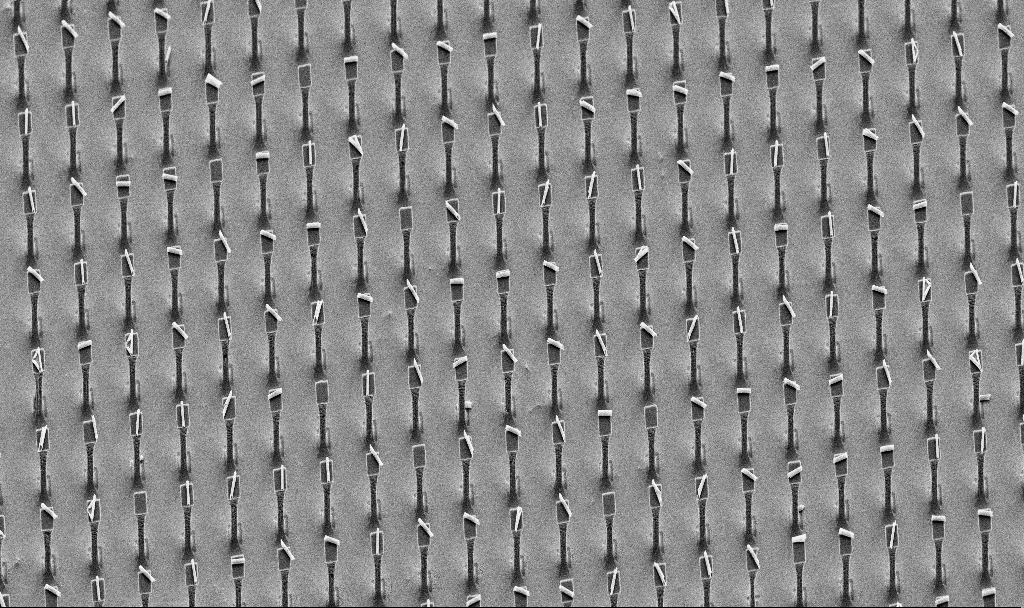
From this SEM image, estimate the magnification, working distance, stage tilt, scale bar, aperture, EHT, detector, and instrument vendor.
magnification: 1.42 K X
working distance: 9.5 mm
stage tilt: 30°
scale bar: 10000 nm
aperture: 30 µm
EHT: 5 kV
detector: SE2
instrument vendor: Zeiss